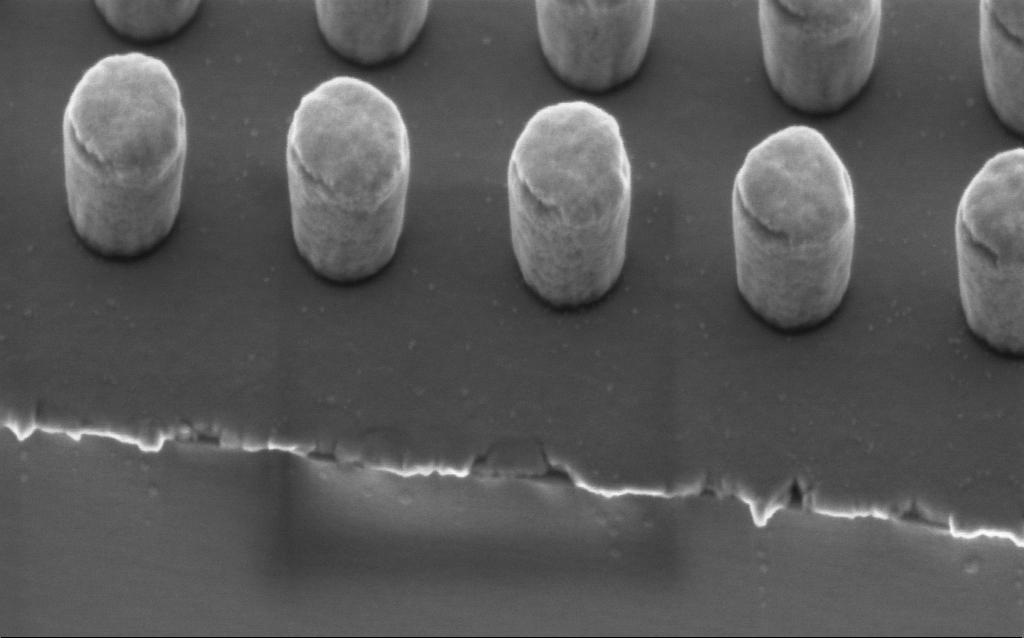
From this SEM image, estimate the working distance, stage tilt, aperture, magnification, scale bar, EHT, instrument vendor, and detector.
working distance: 3 mm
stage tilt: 45°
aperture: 30 µm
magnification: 165.91 K X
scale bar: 200 nm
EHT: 2 kV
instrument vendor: Zeiss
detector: InLens